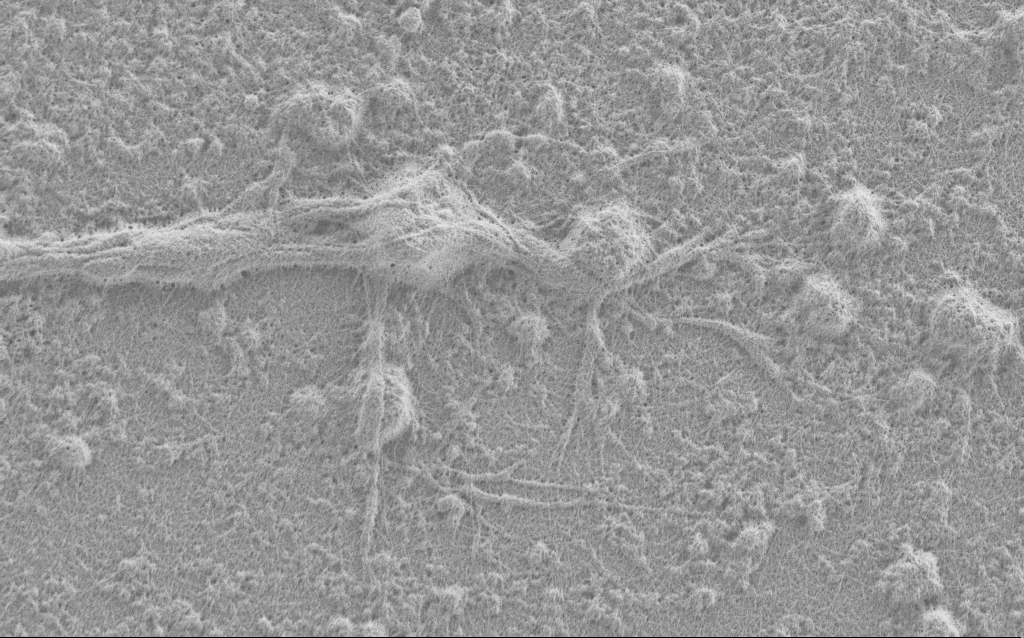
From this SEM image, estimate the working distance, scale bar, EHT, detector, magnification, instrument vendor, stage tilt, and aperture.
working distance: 4 mm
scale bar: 2000 nm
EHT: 0.9 kV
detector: SE2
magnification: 10 K X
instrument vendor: Zeiss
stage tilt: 0°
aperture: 30 µm